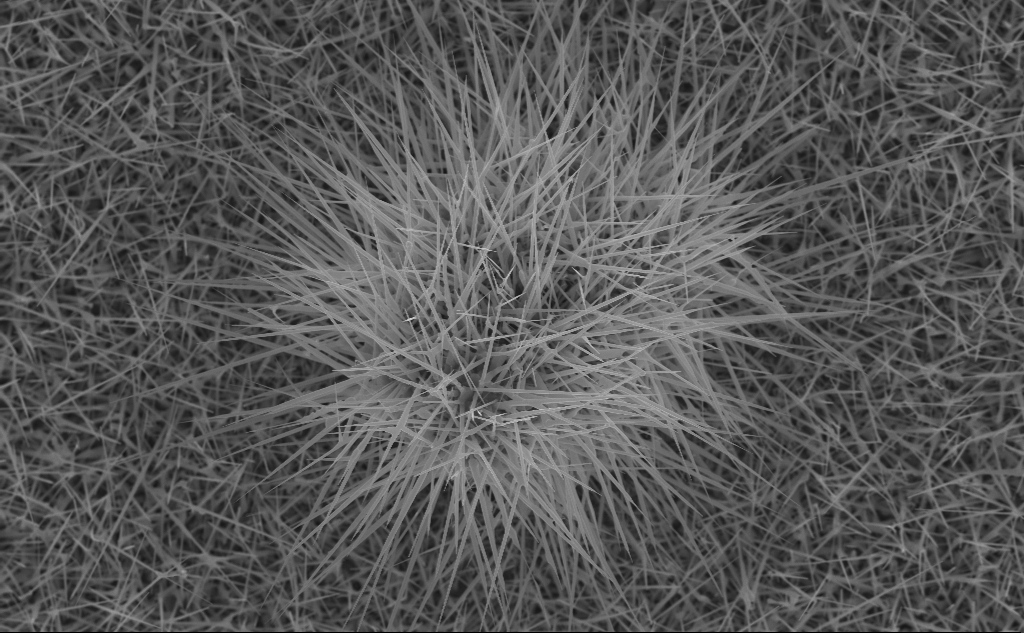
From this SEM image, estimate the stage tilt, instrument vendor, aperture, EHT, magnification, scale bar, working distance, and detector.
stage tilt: -0°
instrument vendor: Zeiss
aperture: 30 µm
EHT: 10 kV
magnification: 13.9 K X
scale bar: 2000 nm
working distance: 7 mm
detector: InLens